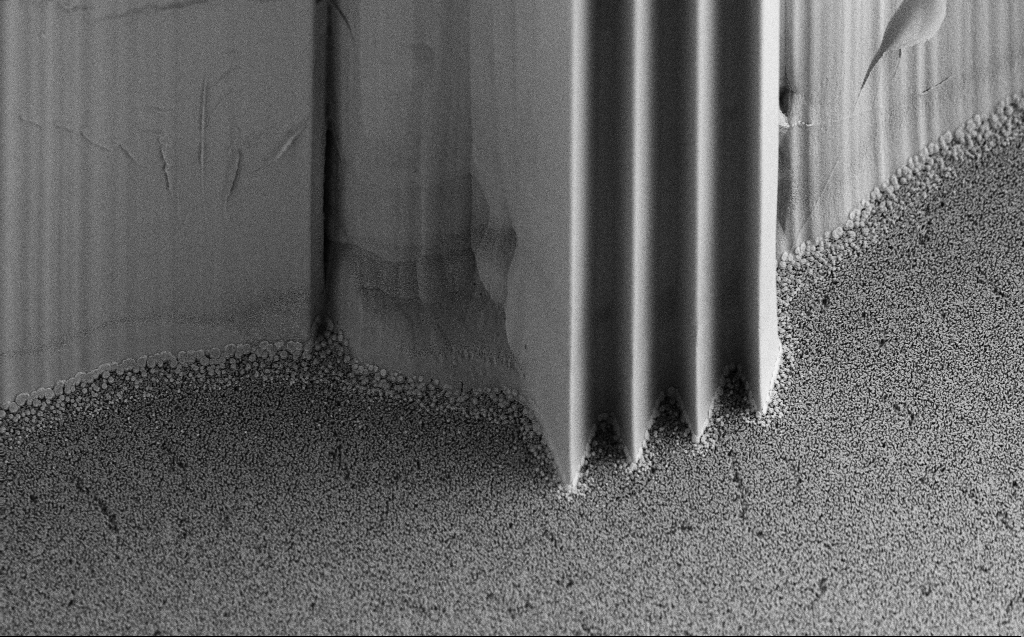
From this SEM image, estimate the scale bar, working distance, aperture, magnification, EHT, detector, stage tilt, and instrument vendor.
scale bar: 10000 nm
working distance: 5 mm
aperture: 30 µm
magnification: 2.18 K X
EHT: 5 kV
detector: SE2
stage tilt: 45°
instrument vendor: Zeiss